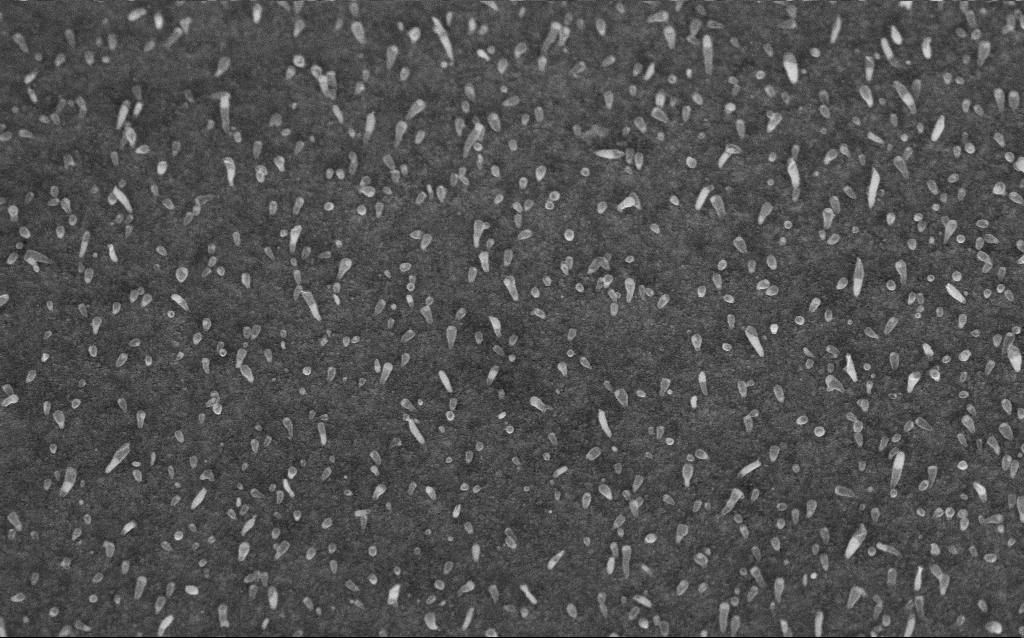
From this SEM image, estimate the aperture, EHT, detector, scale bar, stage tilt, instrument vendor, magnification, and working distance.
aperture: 30 µm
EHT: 5 kV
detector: InLens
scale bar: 1000 nm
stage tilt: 45°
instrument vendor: Zeiss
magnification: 50 K X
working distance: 7.2 mm